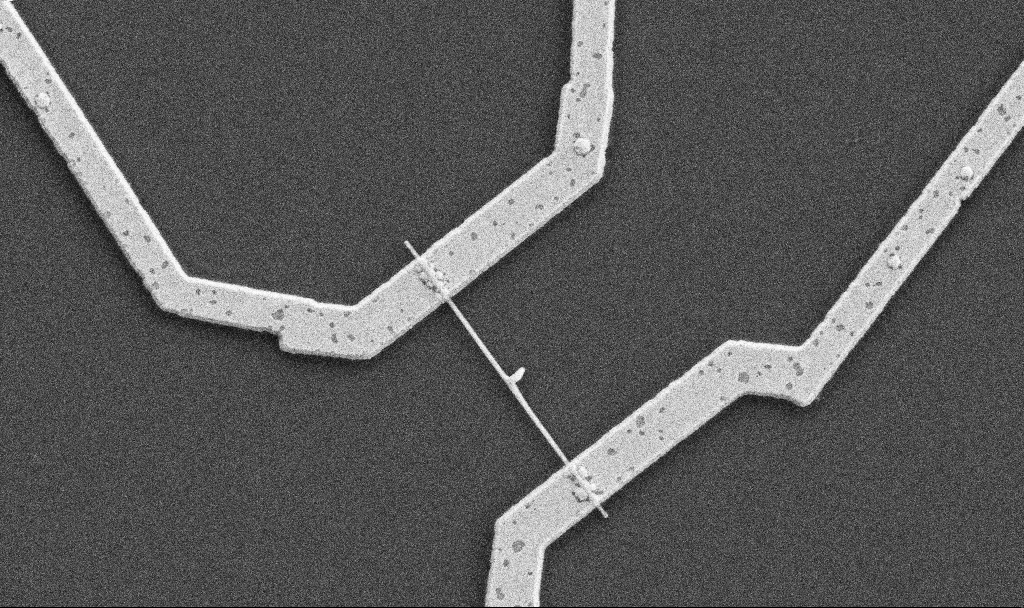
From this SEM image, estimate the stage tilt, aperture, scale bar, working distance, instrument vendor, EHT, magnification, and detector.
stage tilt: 0°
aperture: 30 µm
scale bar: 1000 nm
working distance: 10.7 mm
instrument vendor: Zeiss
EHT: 5 kV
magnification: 20 K X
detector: SE2